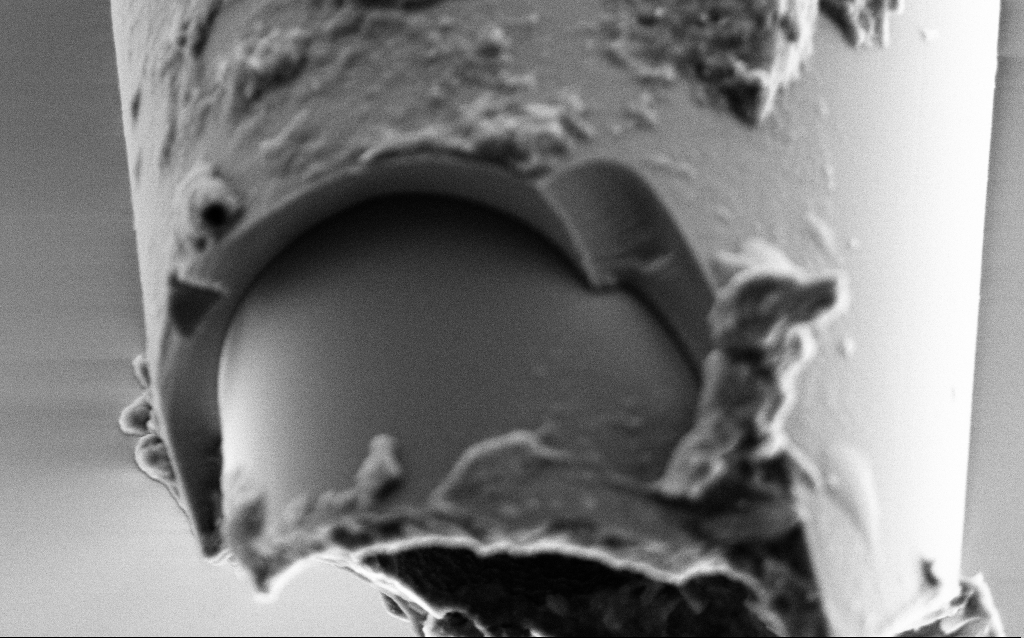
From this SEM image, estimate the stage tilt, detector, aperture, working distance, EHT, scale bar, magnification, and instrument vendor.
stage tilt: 45°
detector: SE2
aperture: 30 µm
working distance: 6 mm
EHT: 2 kV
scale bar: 1000 nm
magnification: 50 K X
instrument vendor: Zeiss